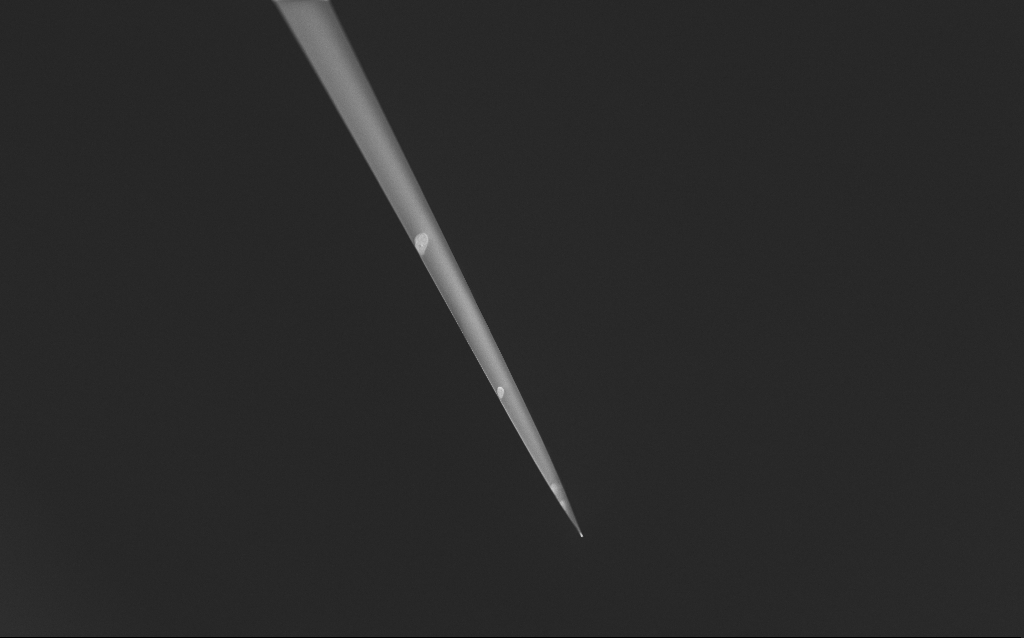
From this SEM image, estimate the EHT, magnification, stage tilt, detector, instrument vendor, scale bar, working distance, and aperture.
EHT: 1 kV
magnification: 0.34 K X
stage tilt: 45°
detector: InLens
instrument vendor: Zeiss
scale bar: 200000 nm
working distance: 6 mm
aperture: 30 µm